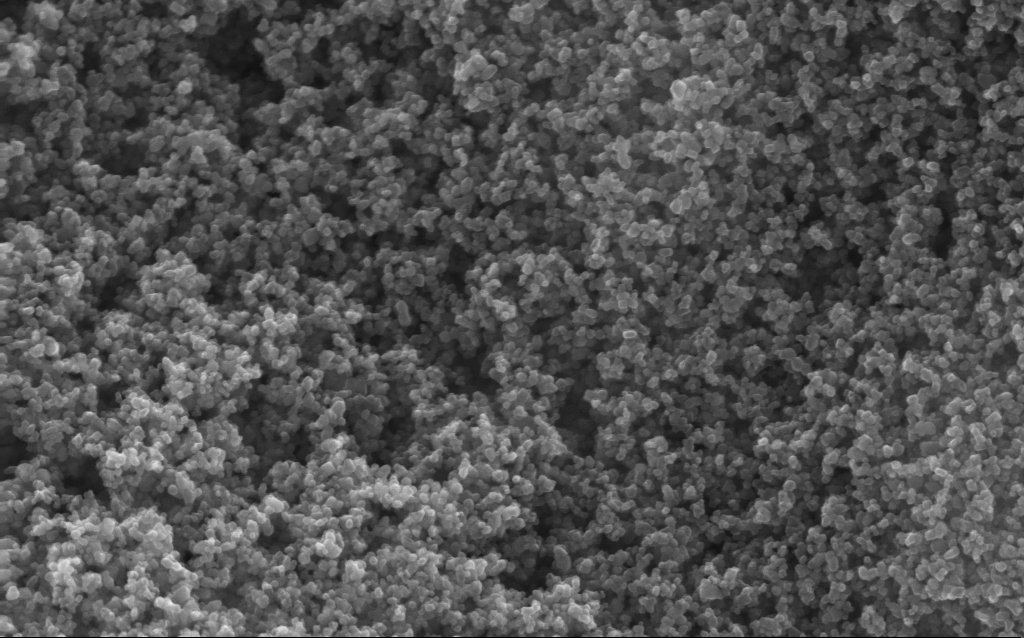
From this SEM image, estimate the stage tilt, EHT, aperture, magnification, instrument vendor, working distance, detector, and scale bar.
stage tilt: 0°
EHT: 10 kV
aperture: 30 µm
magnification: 130 K X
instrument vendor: Zeiss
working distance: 2.6 mm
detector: InLens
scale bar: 100 nm